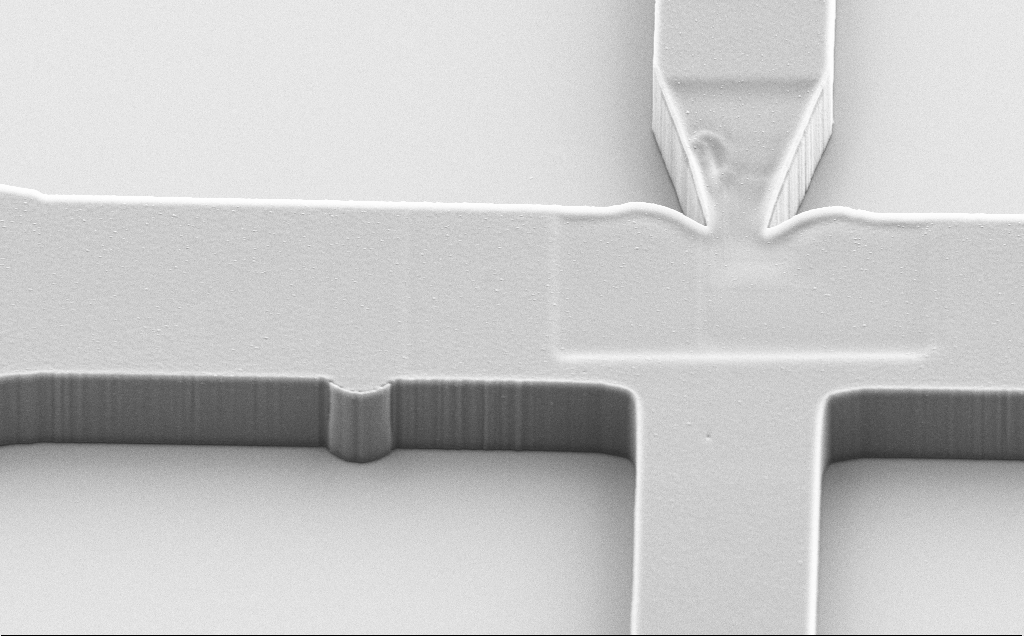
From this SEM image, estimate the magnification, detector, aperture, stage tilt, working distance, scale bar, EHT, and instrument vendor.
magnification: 6.52 K X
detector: SE2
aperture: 30 µm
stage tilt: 45°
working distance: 9 mm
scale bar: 10000 nm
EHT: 5 kV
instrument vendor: Zeiss